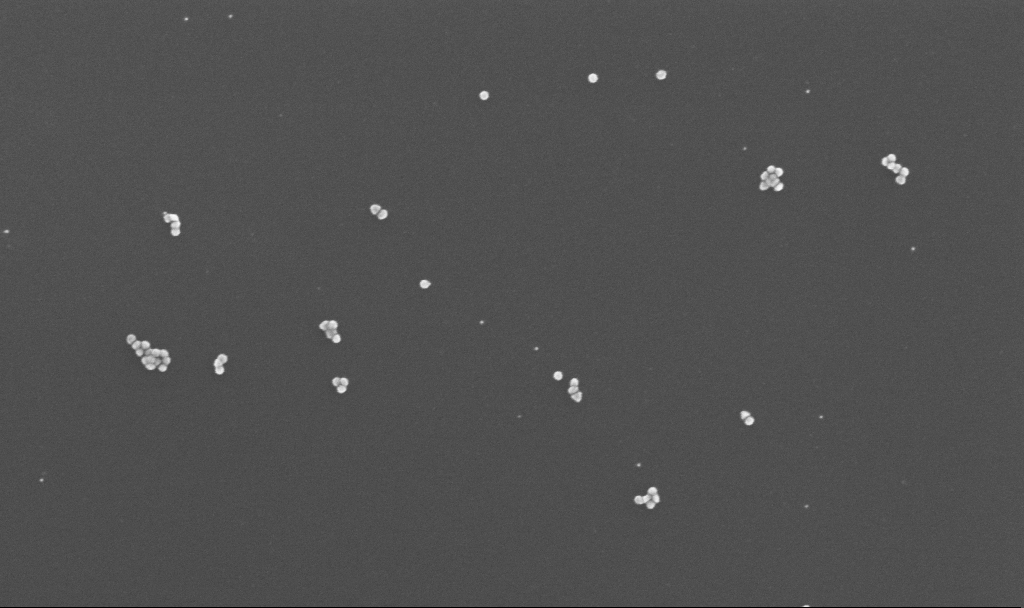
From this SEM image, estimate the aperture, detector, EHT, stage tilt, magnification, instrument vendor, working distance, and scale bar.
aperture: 30 µm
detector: InLens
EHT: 10 kV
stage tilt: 0°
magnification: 150 K X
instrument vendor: Zeiss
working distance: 3.4 mm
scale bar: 100 nm